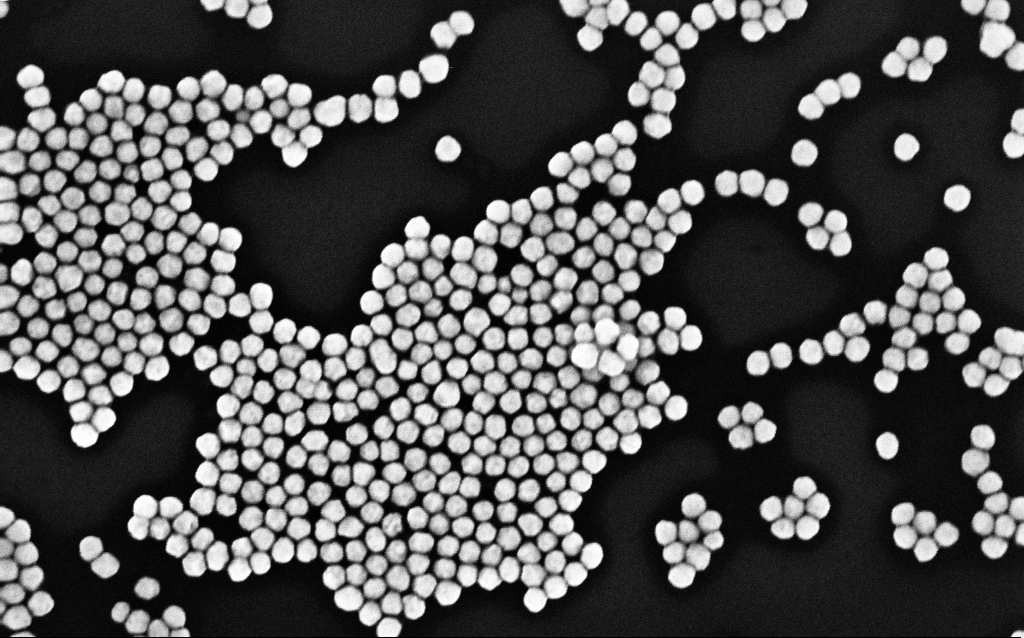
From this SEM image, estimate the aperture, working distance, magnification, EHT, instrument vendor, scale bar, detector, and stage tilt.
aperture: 30 µm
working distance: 3.1 mm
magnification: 143.25 K X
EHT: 10 kV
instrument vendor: Zeiss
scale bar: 200 nm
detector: InLens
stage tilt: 0°